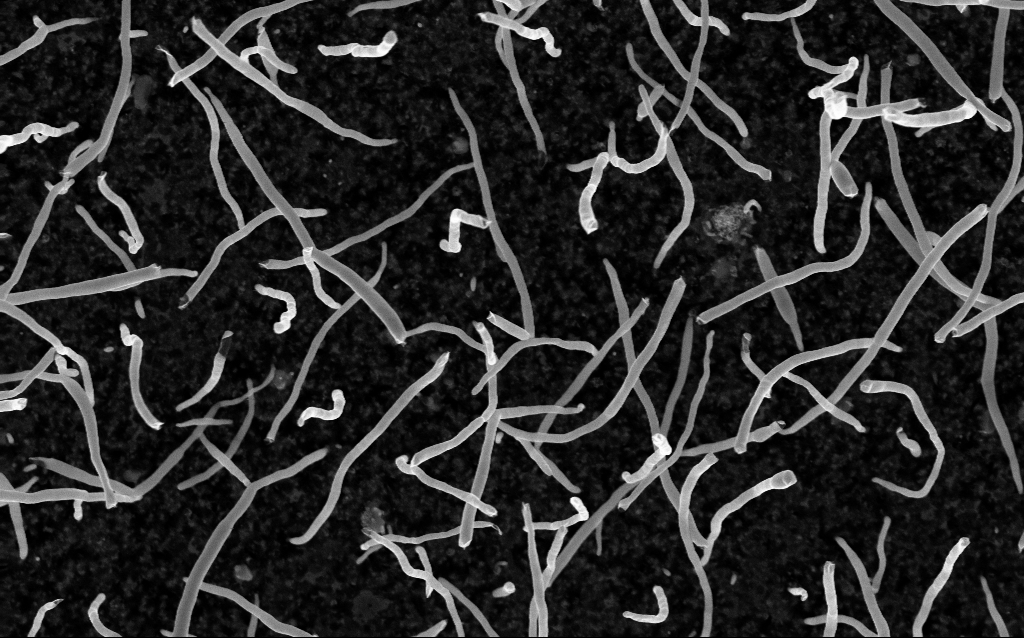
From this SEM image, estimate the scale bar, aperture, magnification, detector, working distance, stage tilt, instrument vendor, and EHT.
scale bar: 1000 nm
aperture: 30 µm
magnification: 50 K X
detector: InLens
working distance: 2.1 mm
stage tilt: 0°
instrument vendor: Zeiss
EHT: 5 kV